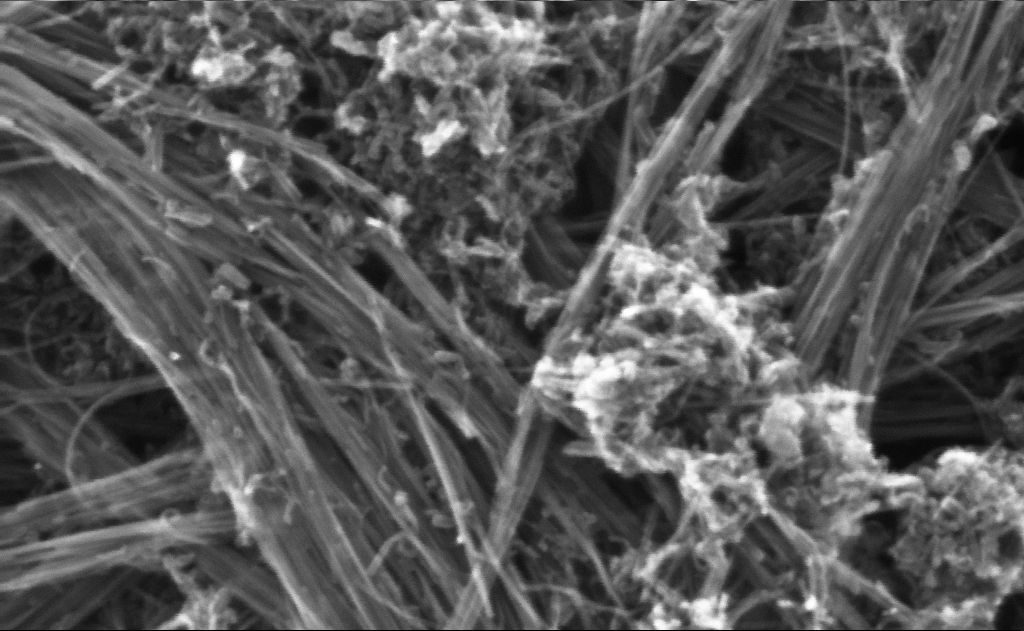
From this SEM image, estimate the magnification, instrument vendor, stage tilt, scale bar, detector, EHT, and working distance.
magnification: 528.2 K X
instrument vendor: Zeiss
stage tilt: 0°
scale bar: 100 nm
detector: InLens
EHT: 10 kV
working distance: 3 mm